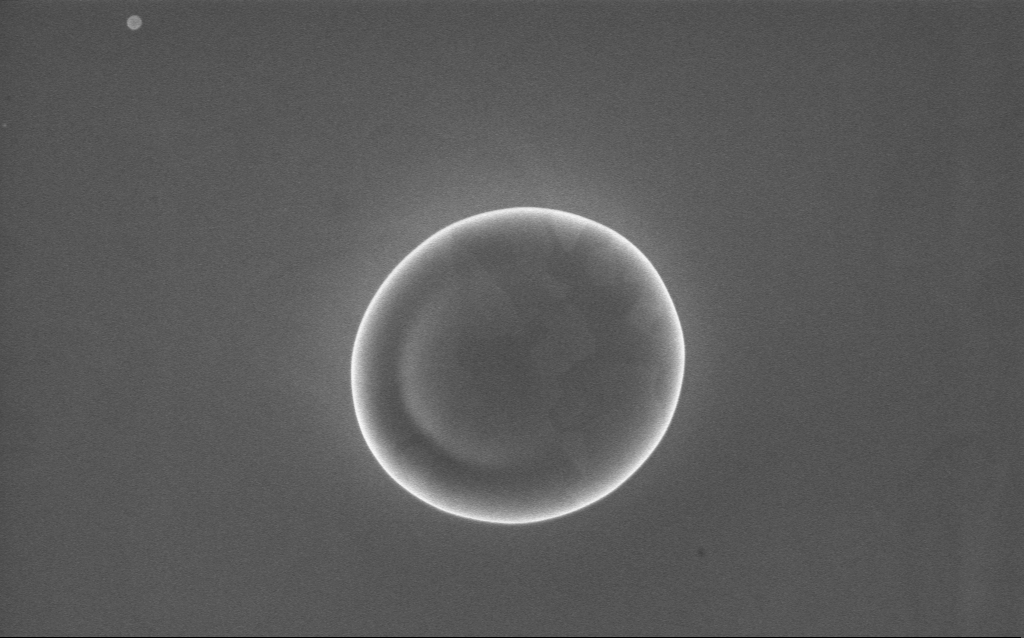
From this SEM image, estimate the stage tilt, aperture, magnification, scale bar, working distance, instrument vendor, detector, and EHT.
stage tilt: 0°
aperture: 30 µm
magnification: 36 K X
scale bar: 1000 nm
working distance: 2 mm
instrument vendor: Zeiss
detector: InLens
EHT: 10 kV